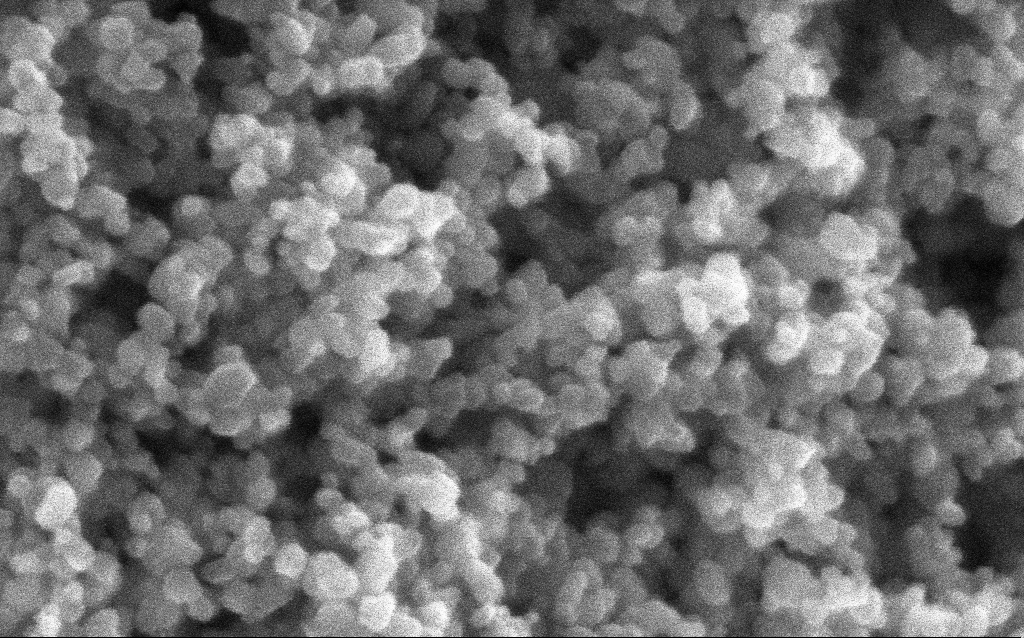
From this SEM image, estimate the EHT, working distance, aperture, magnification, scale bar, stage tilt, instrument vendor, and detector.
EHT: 5 kV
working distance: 2.9 mm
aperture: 30 µm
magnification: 416 K X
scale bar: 100 nm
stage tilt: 0°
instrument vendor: Zeiss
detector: InLens